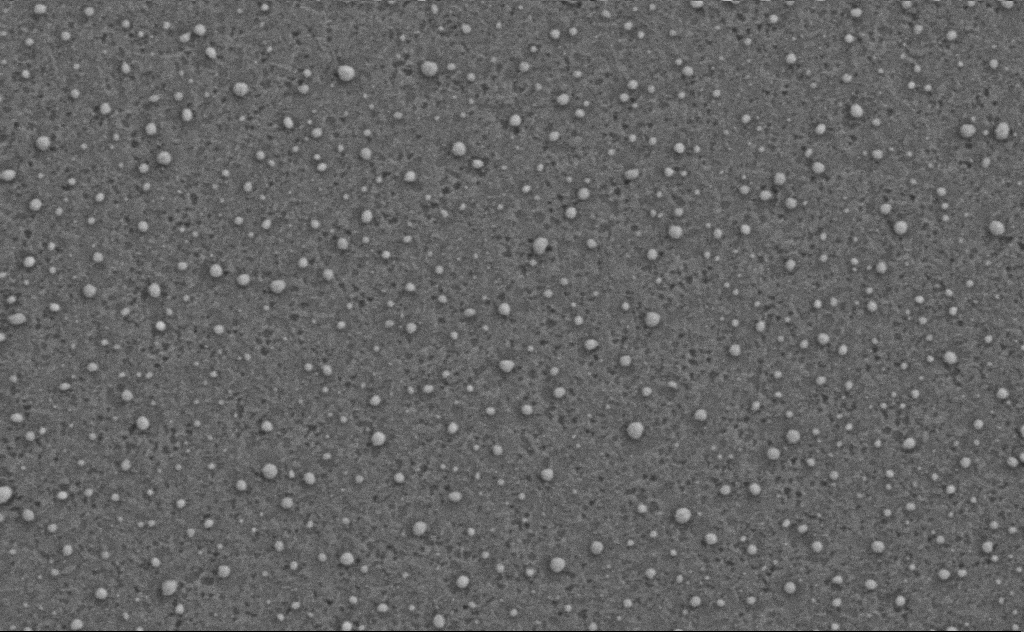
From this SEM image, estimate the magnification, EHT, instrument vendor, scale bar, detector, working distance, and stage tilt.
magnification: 80 K X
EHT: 3 kV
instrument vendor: Zeiss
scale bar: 200 nm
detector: SE2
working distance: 4 mm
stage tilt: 0°